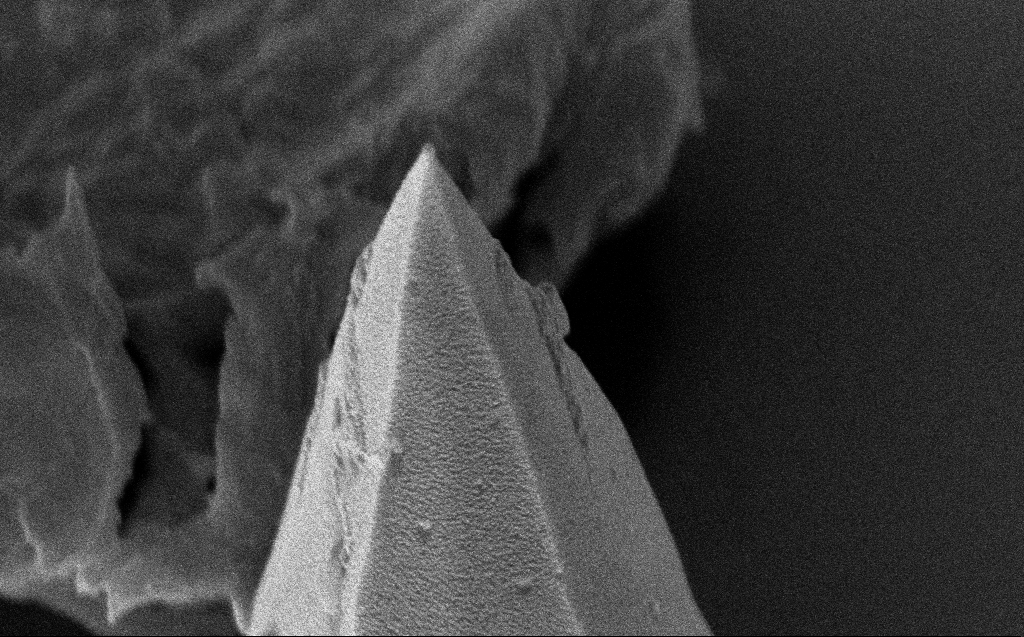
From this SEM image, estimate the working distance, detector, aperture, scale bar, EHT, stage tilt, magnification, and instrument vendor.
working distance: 7 mm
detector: InLens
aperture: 30 µm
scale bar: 1000 nm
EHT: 4 kV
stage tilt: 24.8°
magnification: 56.14 K X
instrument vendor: Zeiss